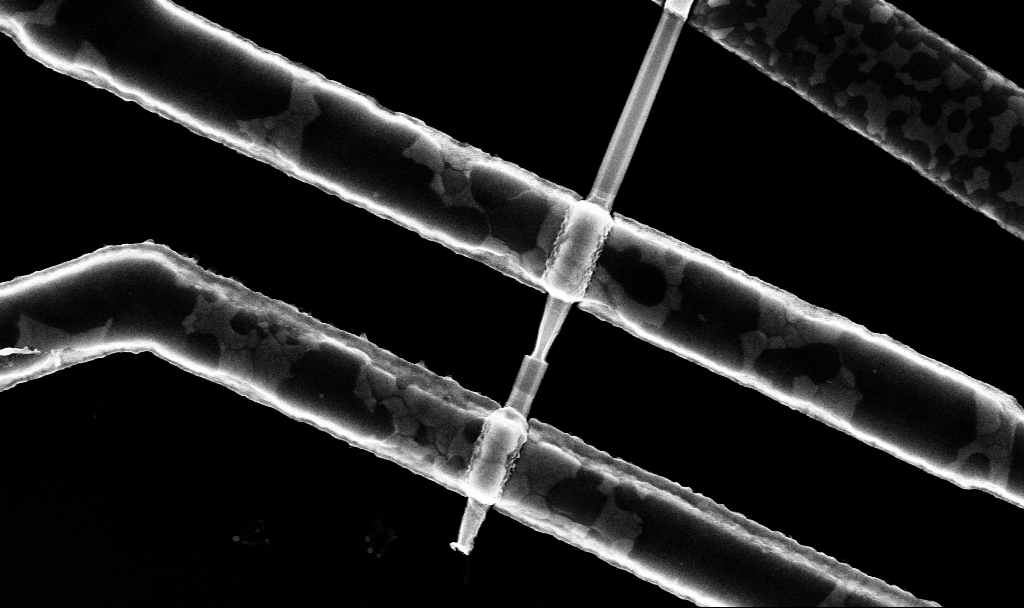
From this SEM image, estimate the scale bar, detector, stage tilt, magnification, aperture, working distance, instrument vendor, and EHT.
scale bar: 1000 nm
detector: InLens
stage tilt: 0°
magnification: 64 K X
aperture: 30 µm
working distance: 7.7 mm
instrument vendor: Zeiss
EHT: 10 kV